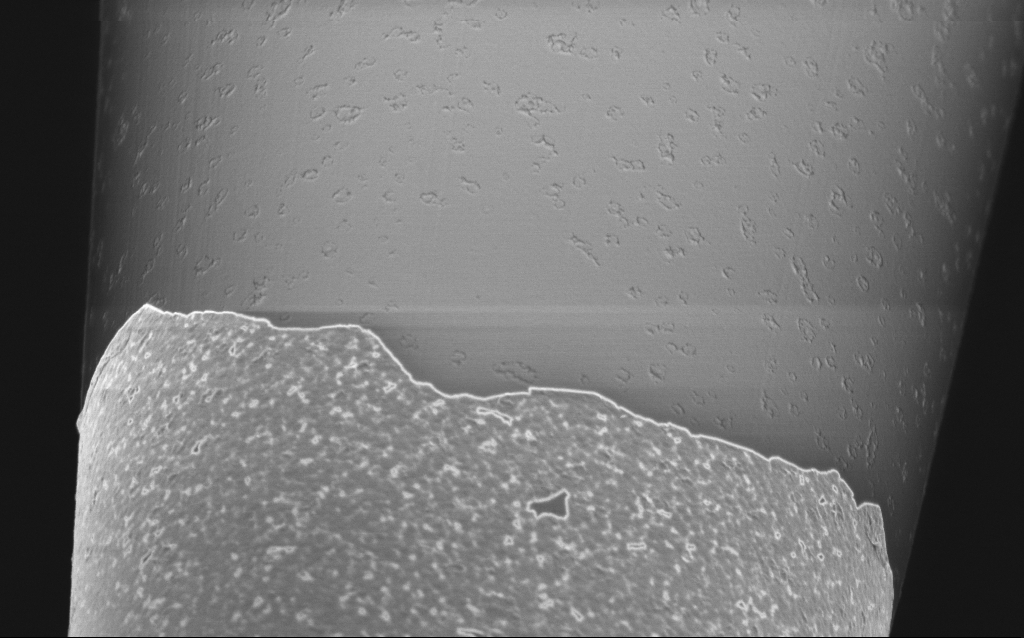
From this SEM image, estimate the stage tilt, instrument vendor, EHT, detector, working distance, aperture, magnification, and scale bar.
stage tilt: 45°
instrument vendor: Zeiss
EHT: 1 kV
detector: InLens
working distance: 6 mm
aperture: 30 µm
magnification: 25 K X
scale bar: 1000 nm